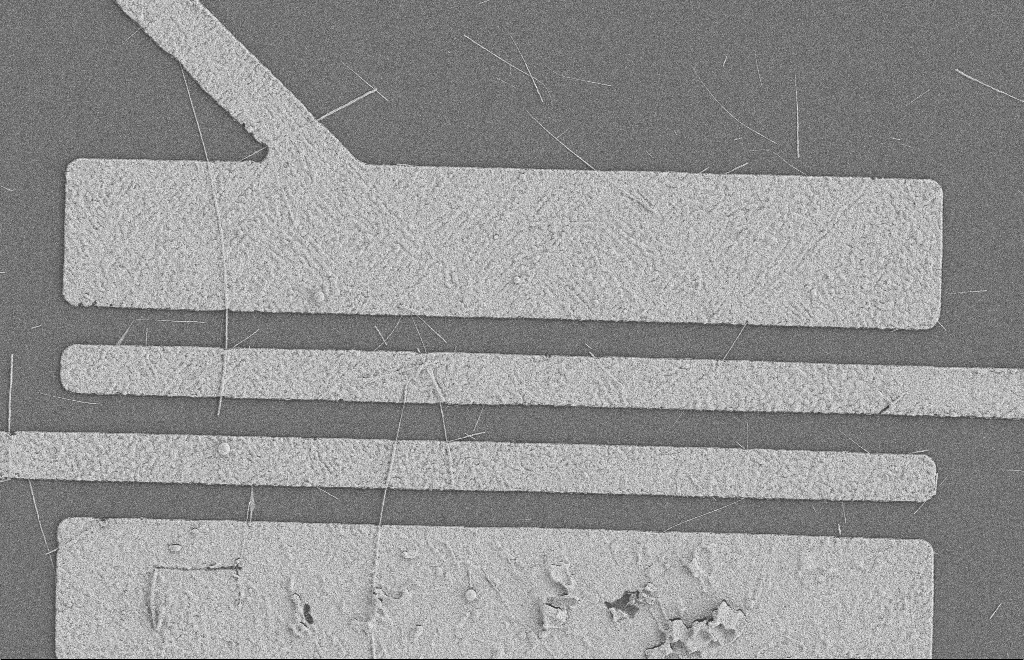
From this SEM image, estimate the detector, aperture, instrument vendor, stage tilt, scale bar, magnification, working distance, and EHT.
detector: SE2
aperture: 20 µm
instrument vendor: Zeiss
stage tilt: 0°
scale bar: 2000 nm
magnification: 5.29 K X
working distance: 8 mm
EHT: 2 kV